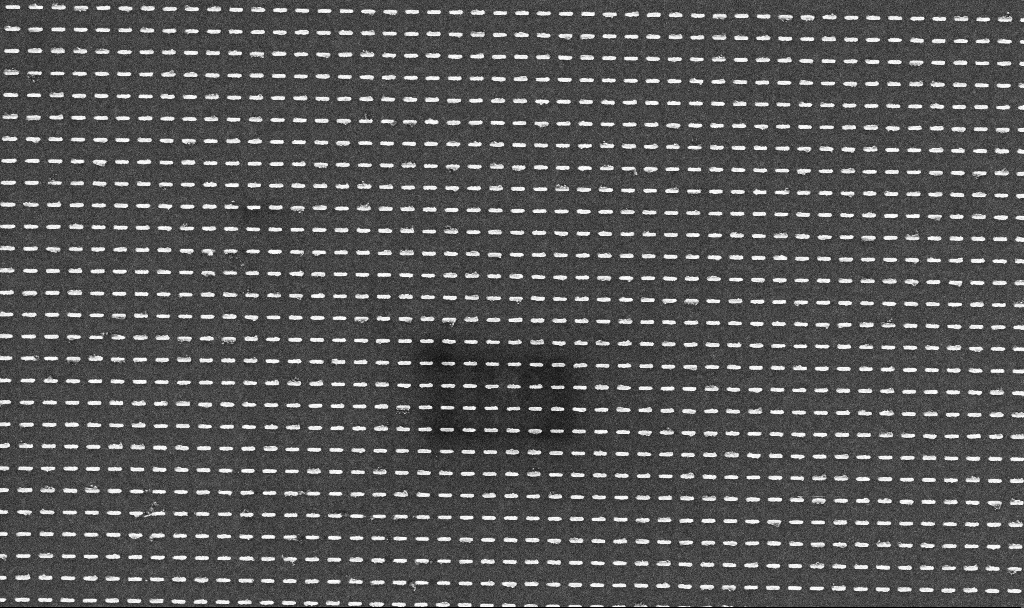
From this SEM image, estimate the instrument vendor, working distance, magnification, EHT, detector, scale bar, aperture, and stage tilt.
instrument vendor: Zeiss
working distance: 3.2 mm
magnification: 35.24 K X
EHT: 5 kV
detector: InLens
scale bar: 1000 nm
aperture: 30 µm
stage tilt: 0°